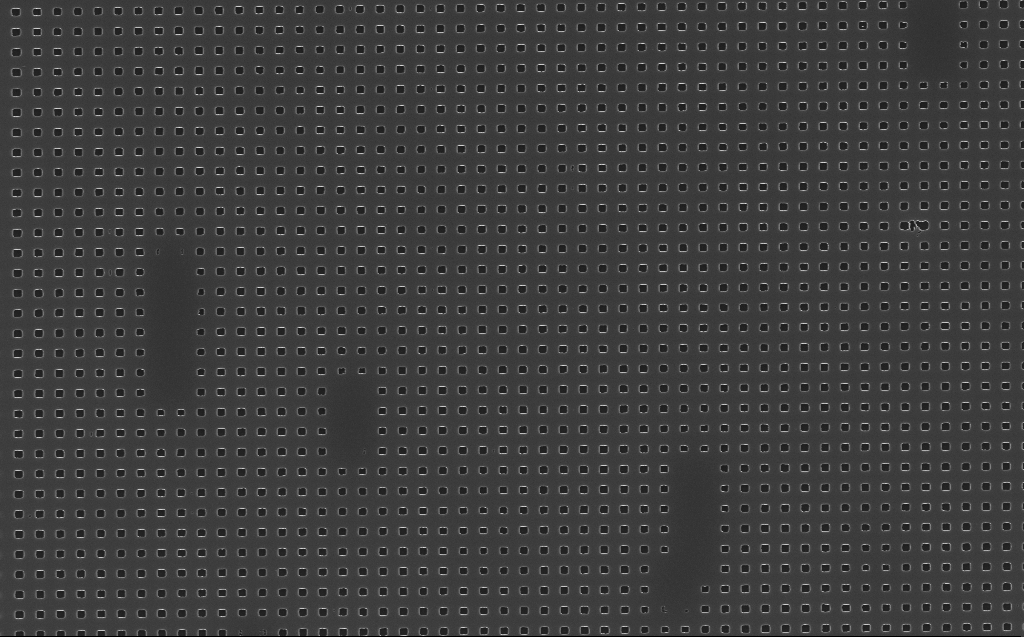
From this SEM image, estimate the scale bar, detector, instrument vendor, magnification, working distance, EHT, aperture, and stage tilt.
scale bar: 2000 nm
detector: InLens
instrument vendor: Zeiss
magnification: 15 K X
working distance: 6 mm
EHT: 10 kV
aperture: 30 µm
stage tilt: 0°